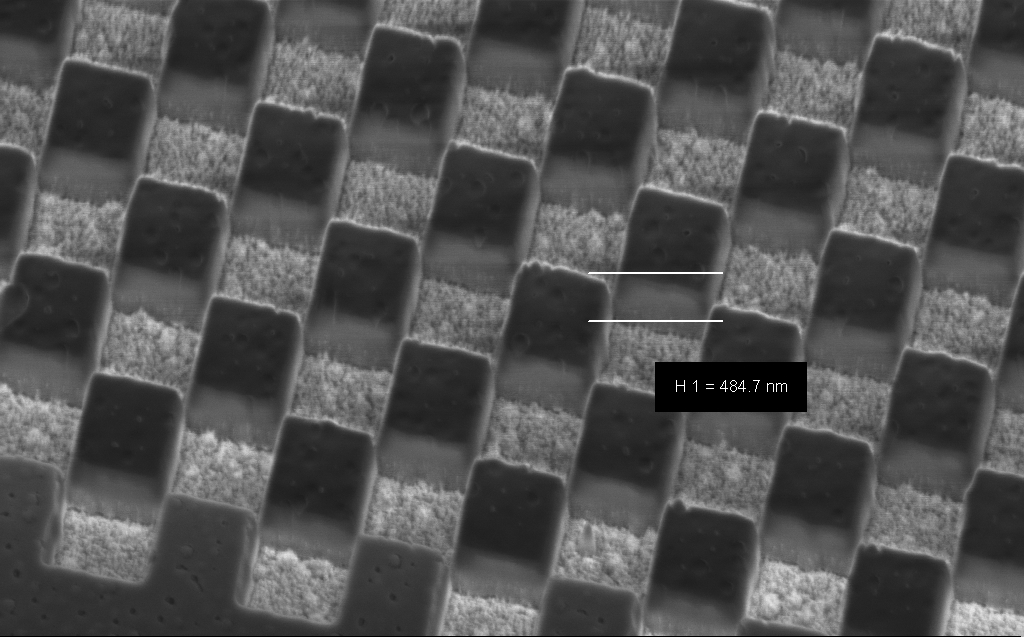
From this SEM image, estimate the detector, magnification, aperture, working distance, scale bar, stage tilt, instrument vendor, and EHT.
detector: InLens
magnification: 36.36 K X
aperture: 30 µm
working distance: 6 mm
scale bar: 1000 nm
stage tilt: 45°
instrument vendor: Zeiss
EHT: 3 kV